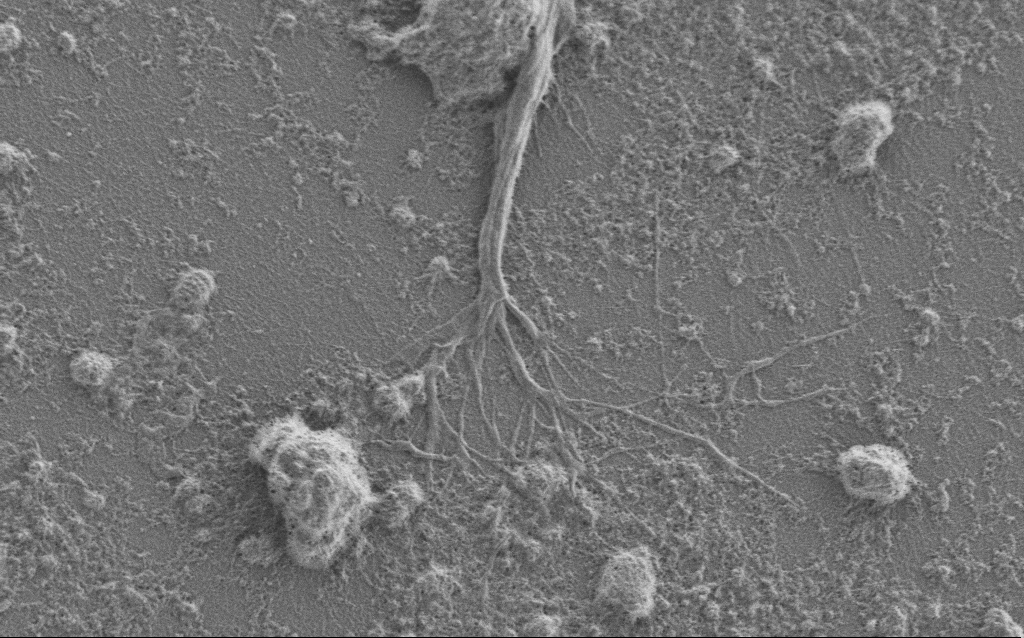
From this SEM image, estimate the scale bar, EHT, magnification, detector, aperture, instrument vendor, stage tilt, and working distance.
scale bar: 2000 nm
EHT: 1 kV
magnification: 7.5 K X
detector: SE2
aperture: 30 µm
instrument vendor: Zeiss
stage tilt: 0°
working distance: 6 mm